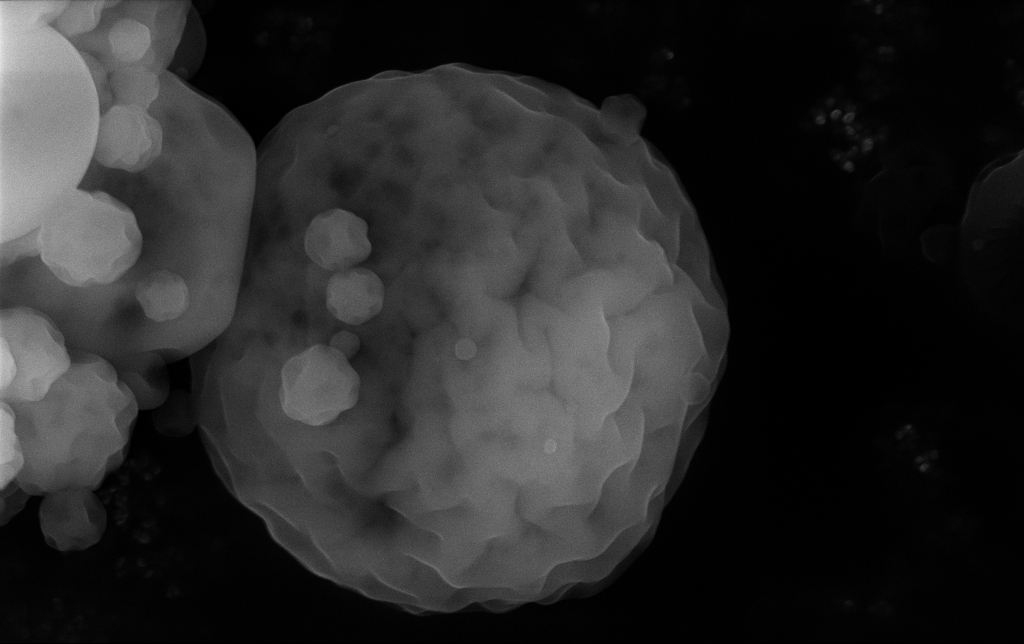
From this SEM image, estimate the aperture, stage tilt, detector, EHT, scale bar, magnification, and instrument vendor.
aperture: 30 µm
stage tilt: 0°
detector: InLens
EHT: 15 kV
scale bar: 1000 nm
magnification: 41.48 K X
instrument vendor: Zeiss